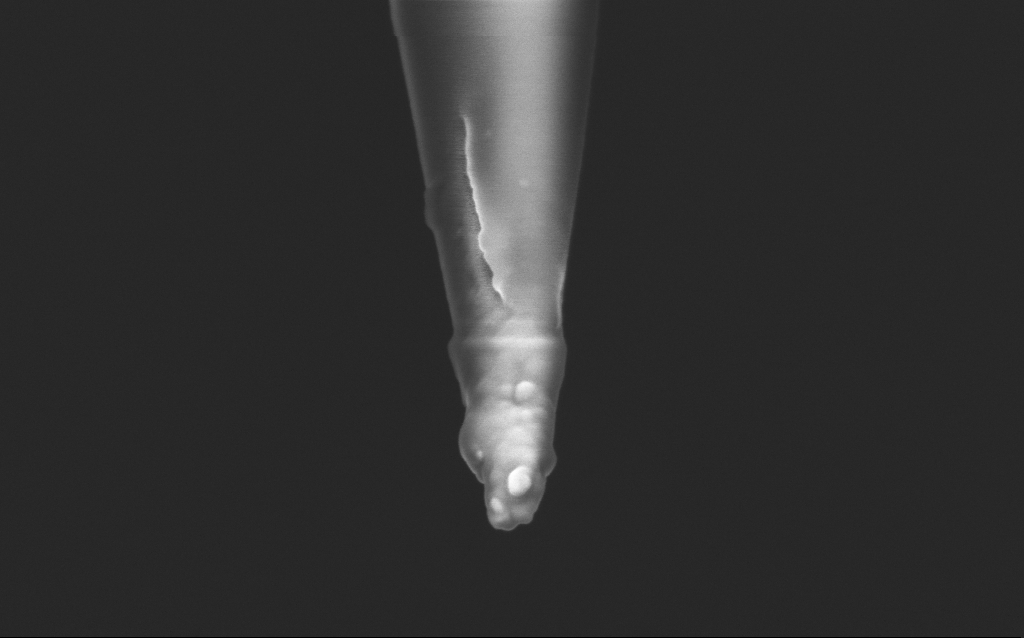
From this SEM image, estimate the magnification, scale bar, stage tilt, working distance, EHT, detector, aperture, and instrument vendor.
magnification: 100 K X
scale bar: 200 nm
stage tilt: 45°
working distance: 5 mm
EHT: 2.5 kV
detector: InLens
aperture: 30 µm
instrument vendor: Zeiss